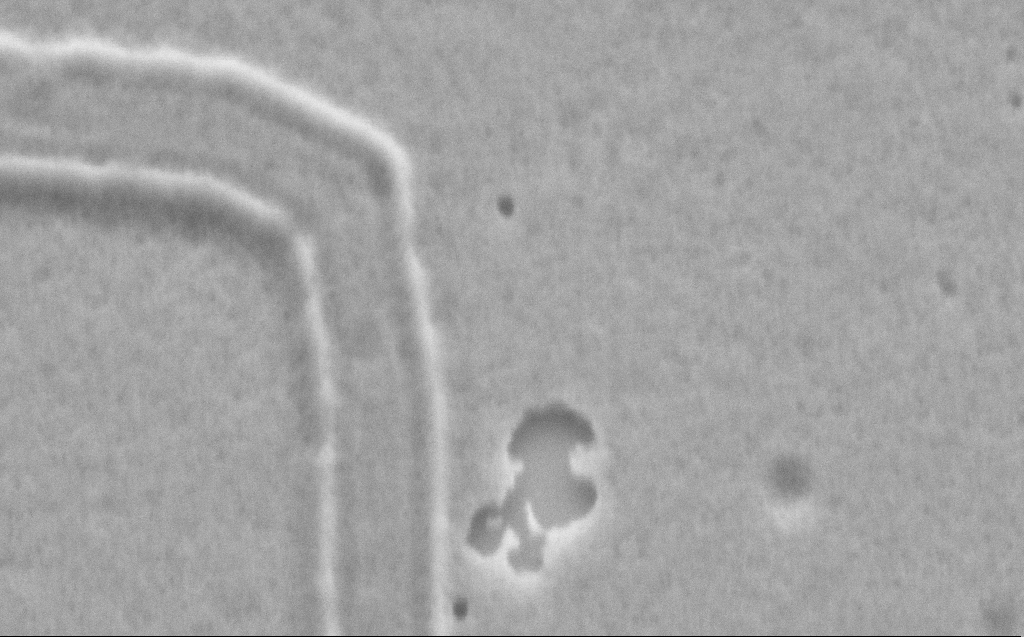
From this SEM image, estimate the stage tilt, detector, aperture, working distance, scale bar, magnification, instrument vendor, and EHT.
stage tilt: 41.9°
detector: SE2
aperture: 30 µm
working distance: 9 mm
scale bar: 200 nm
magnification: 101.63 K X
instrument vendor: Zeiss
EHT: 3 kV